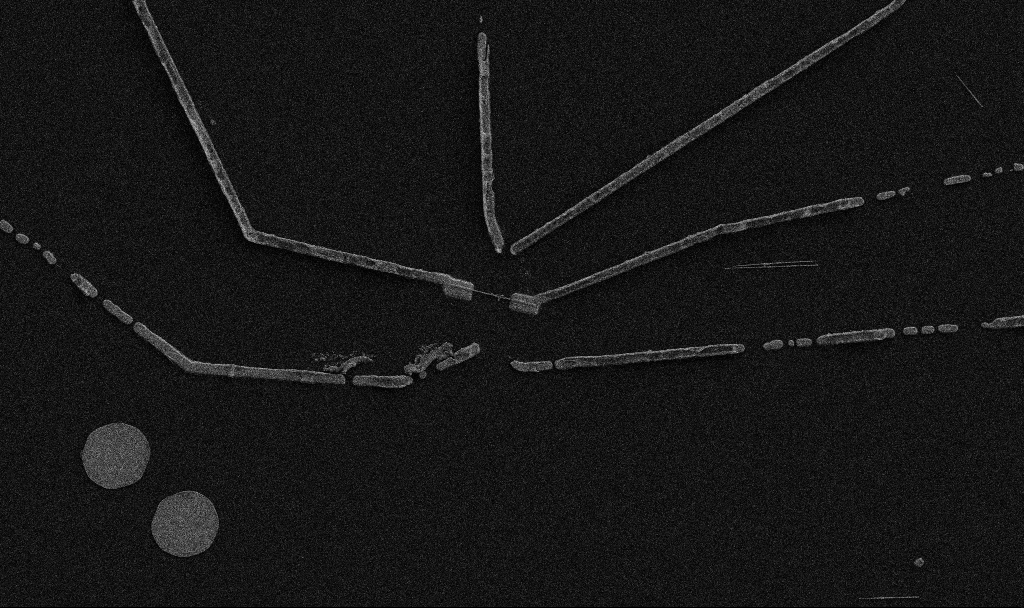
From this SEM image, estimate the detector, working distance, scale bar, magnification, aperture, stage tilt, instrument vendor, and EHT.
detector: SE2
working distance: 10.7 mm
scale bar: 10000 nm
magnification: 5 K X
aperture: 30 µm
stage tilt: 0°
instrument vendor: Zeiss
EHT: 5 kV